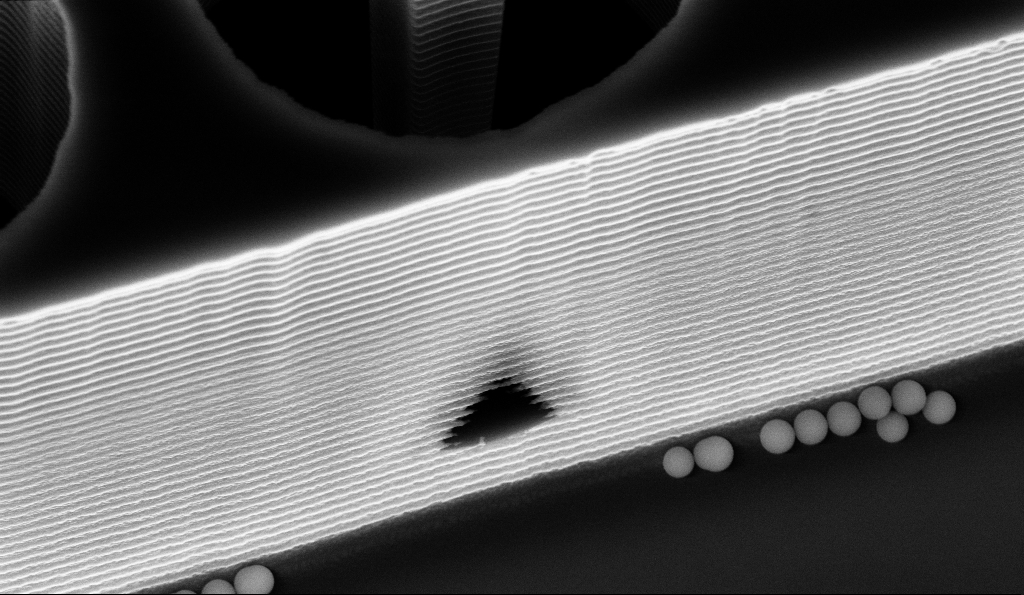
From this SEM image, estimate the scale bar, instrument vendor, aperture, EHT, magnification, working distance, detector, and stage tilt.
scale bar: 1000 nm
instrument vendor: Zeiss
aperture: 30 µm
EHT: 10 kV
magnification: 21.78 K X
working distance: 14.1 mm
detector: InLens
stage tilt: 30°